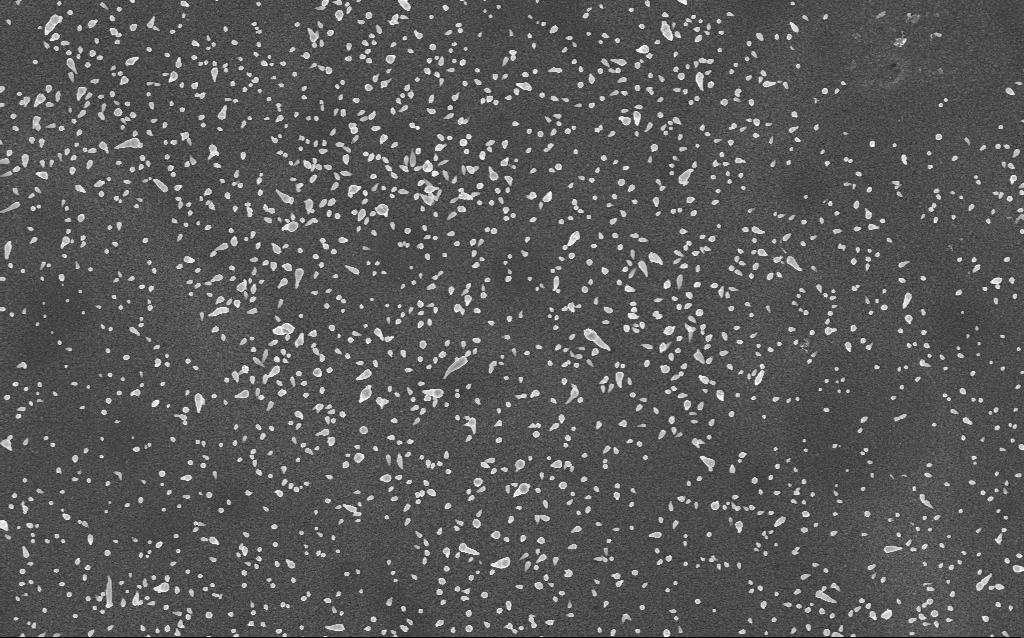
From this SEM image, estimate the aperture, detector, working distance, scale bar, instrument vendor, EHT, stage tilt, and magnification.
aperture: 30 µm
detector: InLens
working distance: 4 mm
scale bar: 2000 nm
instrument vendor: Zeiss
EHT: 5 kV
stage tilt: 0°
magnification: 20 K X